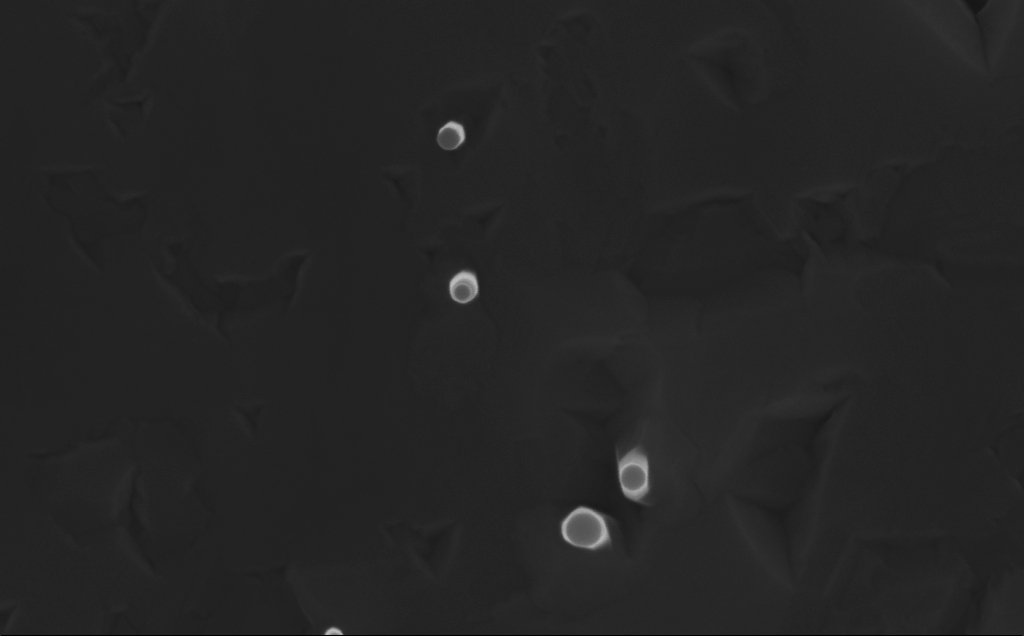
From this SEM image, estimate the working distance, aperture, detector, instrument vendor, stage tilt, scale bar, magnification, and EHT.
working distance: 6 mm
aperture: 30 µm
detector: InLens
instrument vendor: Zeiss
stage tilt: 0°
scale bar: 100 nm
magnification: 150 K X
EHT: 10 kV